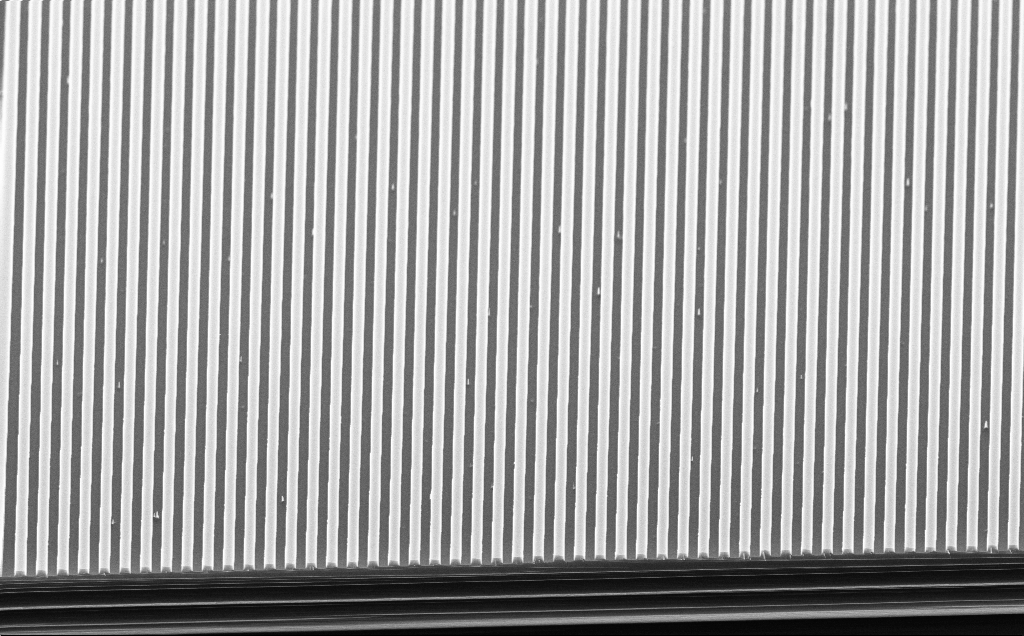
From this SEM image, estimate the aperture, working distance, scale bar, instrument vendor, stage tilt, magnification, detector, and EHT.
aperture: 30 µm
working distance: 4 mm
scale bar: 1000 nm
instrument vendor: Zeiss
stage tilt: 45°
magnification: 15.39 K X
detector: InLens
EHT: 10 kV